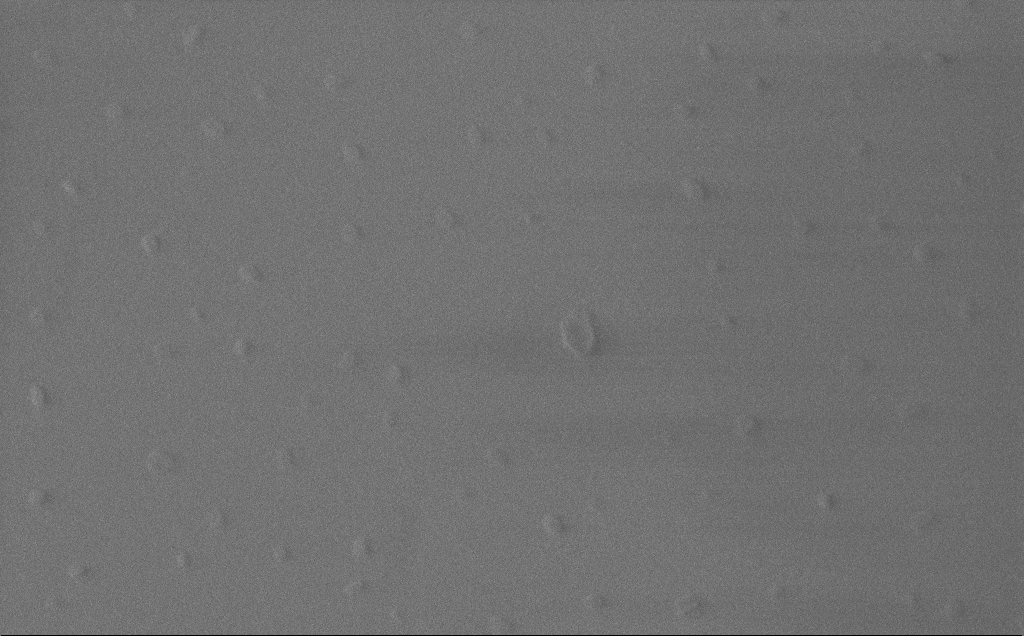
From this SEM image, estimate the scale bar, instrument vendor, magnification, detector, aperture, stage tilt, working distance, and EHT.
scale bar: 200 nm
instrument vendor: Zeiss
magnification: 89.64 K X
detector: InLens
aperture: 30 µm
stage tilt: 0°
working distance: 3 mm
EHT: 1 kV